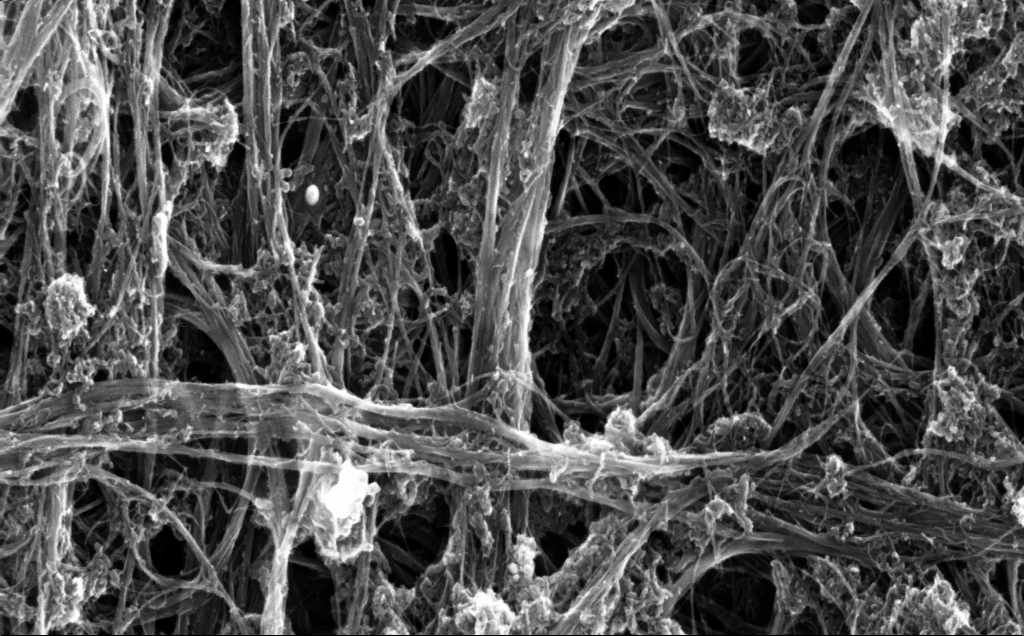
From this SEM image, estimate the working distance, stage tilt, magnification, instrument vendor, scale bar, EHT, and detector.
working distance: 4 mm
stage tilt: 0°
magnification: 138.46 K X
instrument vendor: Zeiss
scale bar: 200 nm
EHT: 5 kV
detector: InLens